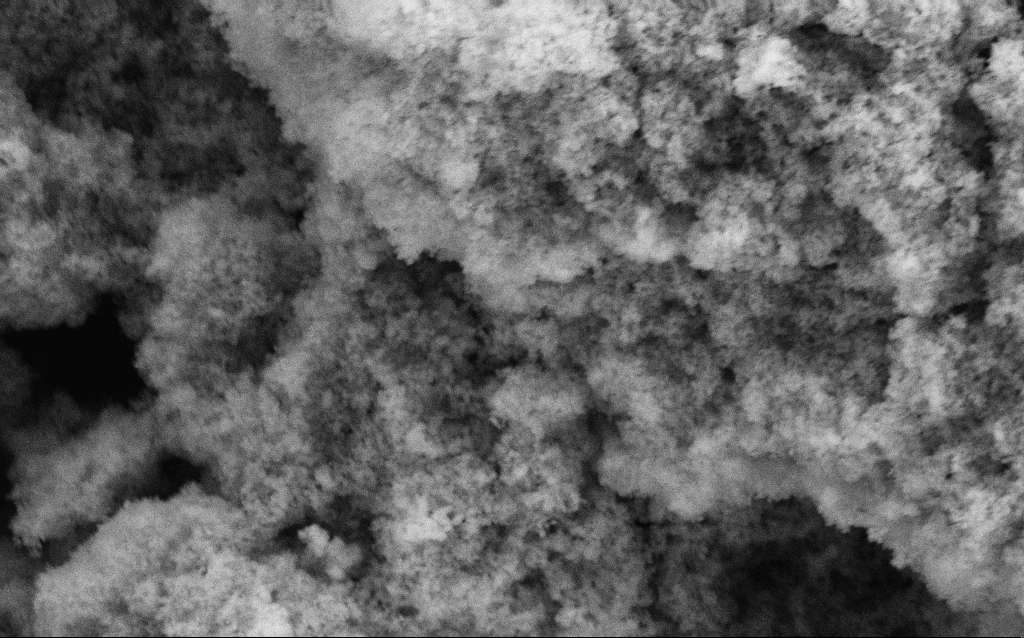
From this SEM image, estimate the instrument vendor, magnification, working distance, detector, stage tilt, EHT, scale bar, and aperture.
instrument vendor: Zeiss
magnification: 68.64 K X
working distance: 4.6 mm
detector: SE2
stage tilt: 0°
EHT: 5 kV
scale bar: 1000 nm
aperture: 30 µm